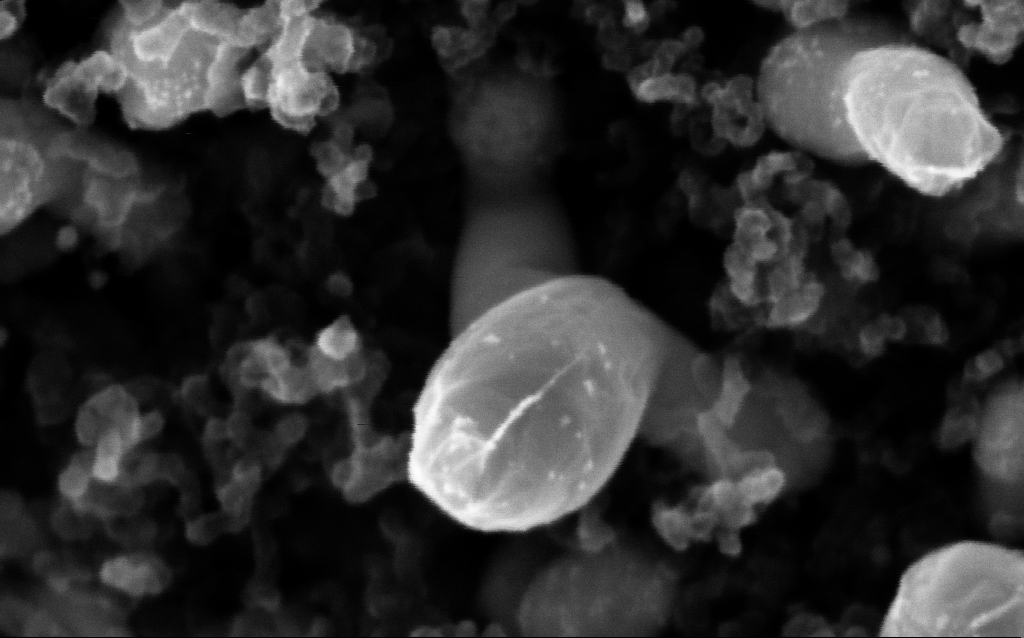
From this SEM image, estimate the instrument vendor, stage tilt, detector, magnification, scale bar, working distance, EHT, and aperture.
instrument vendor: Zeiss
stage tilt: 0°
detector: InLens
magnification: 428.13 K X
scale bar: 100 nm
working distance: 2.3 mm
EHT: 5 kV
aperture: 30 µm